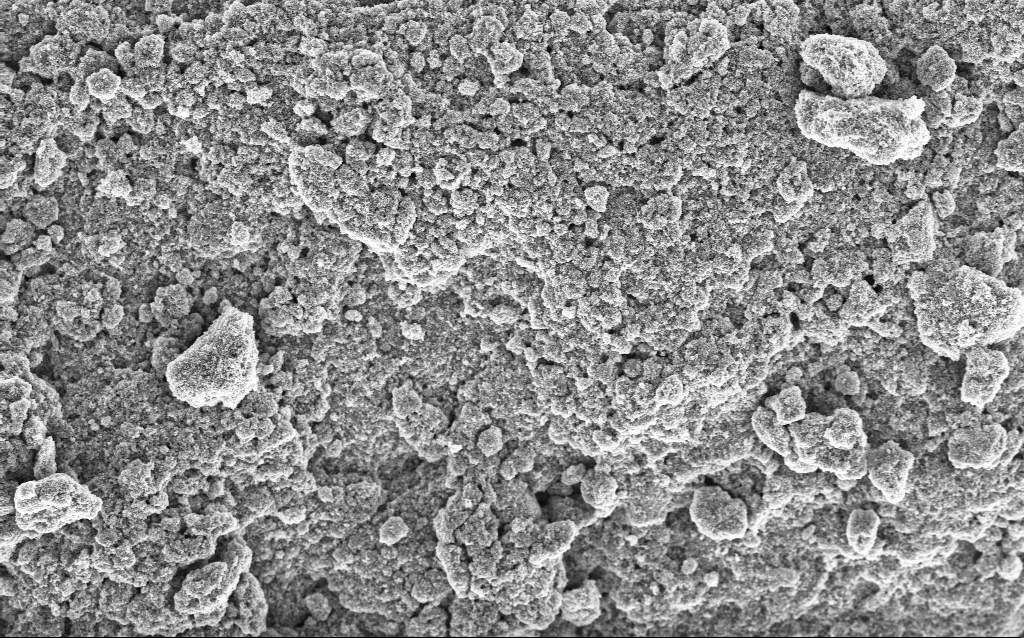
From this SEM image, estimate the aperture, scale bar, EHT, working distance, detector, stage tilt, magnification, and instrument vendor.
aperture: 30 µm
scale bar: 10000 nm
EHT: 5 kV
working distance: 4.5 mm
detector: InLens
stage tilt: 0°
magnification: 6.42 K X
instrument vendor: Zeiss